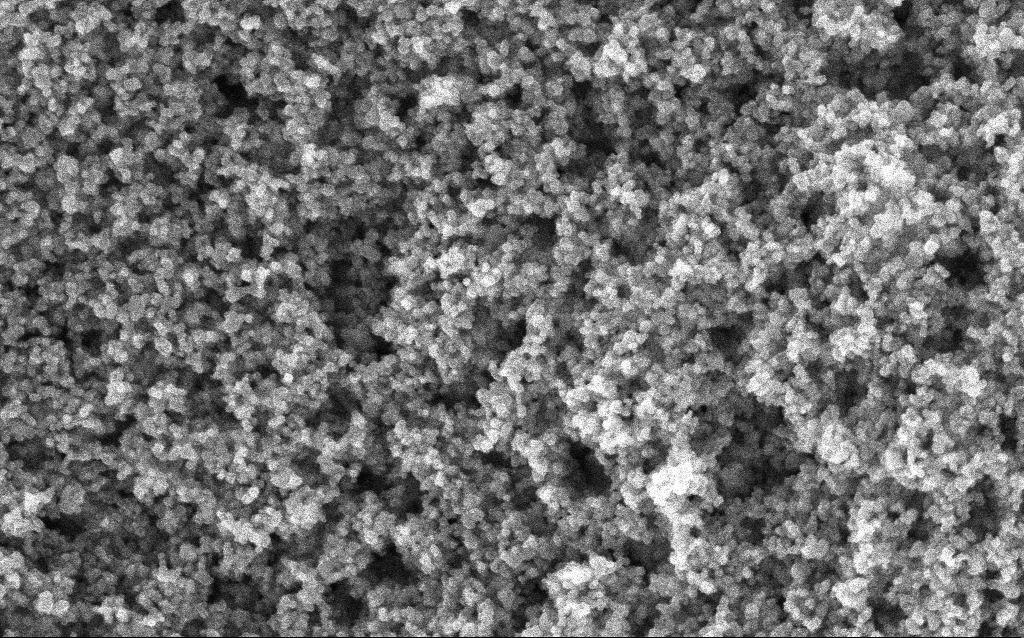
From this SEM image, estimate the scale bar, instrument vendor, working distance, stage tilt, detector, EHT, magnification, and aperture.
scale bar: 100 nm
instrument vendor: Zeiss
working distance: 2.9 mm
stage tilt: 0°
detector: InLens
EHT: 5 kV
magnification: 130 K X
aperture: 30 µm